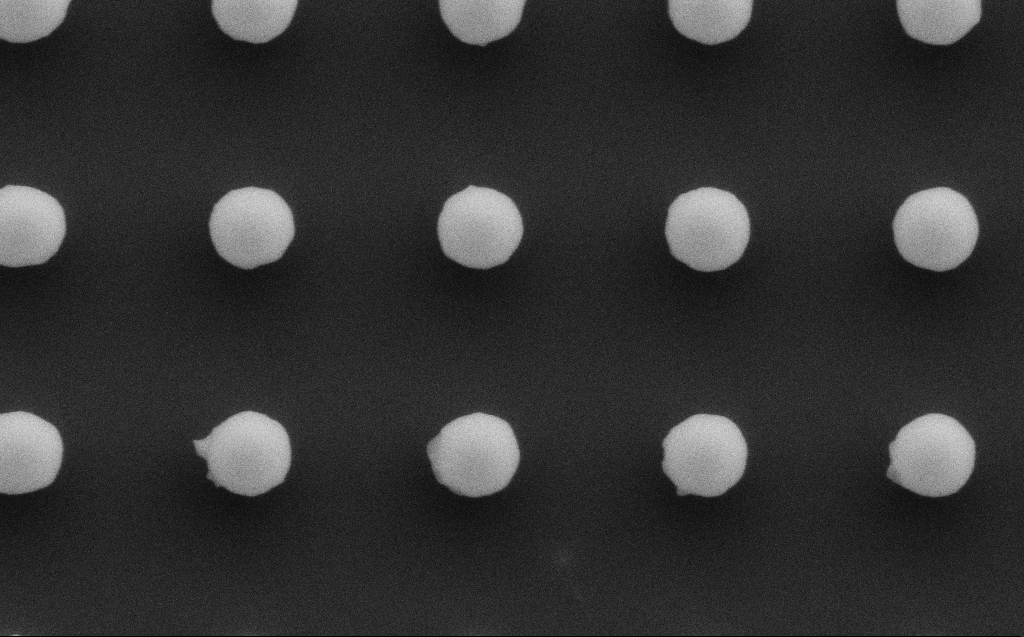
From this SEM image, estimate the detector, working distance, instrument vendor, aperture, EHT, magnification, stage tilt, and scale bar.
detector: SE2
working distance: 6 mm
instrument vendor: Zeiss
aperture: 30 µm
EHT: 5 kV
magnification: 79.45 K X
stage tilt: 0°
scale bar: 200 nm